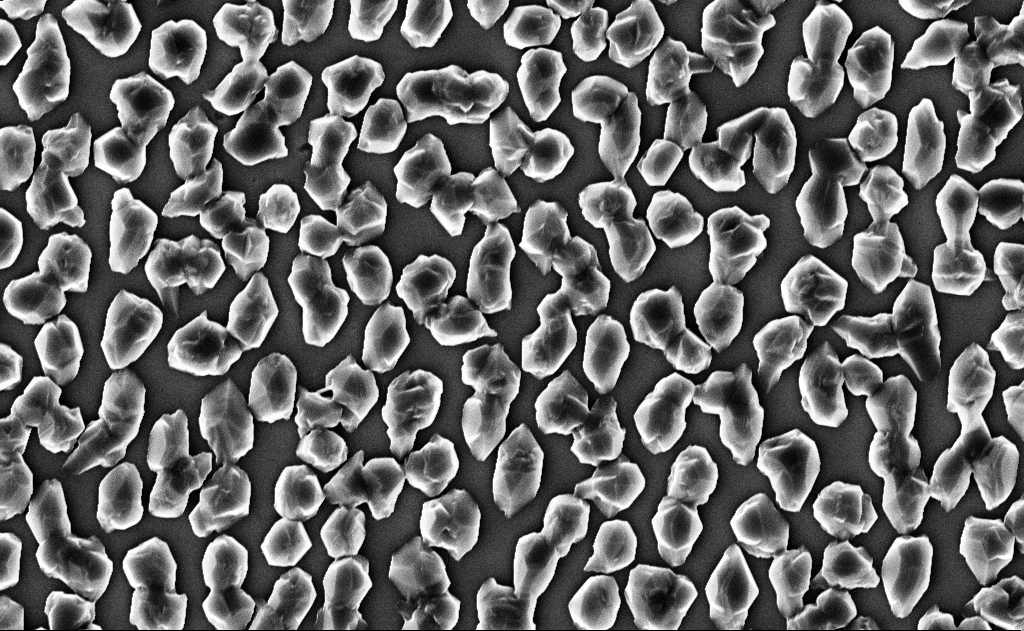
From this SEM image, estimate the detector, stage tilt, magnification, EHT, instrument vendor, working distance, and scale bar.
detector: InLens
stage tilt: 0°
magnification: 20 K X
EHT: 10 kV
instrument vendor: Zeiss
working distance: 12 mm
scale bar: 2000 nm